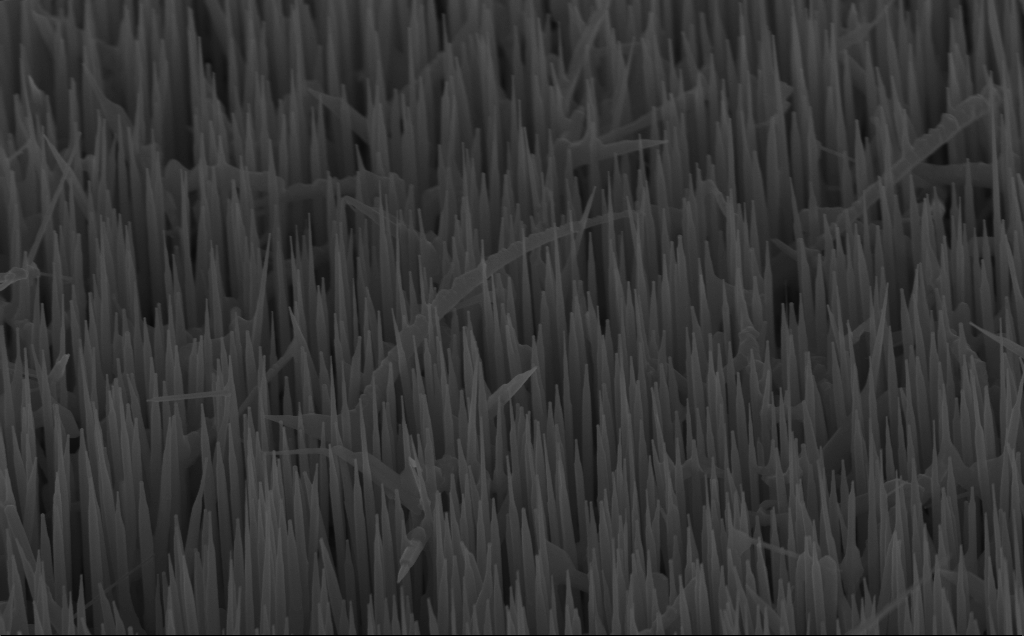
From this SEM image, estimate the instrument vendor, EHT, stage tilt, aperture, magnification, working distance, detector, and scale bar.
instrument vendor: Zeiss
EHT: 10 kV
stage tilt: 45°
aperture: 30 µm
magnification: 40 K X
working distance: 6 mm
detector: InLens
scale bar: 1000 nm